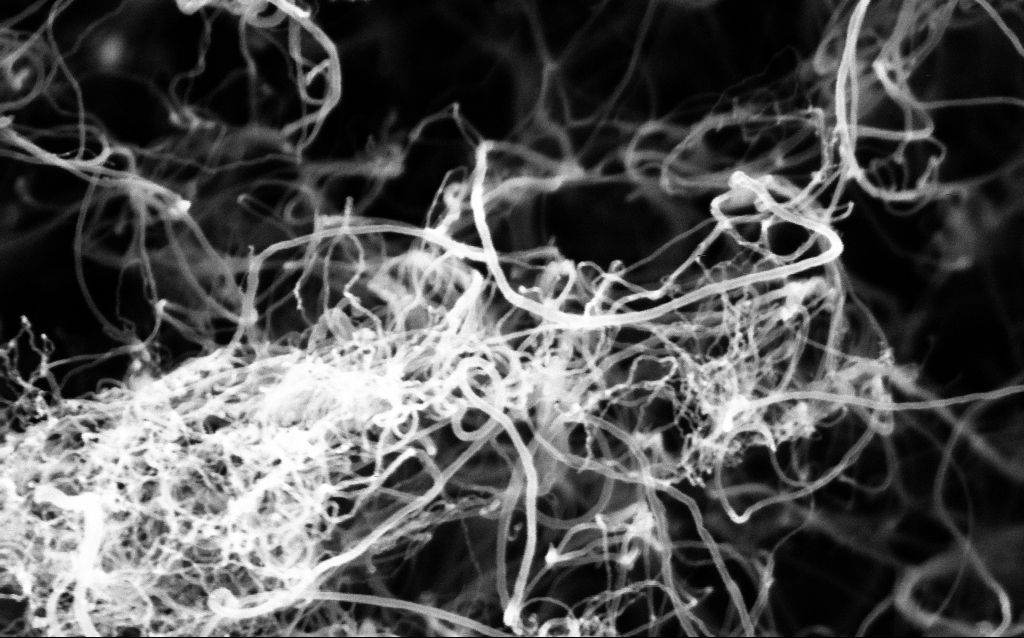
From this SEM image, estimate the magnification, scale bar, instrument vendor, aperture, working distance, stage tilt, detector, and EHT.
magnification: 200 K X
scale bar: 100 nm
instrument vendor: Zeiss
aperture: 30 µm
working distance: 3.3 mm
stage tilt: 0°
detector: InLens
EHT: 5 kV